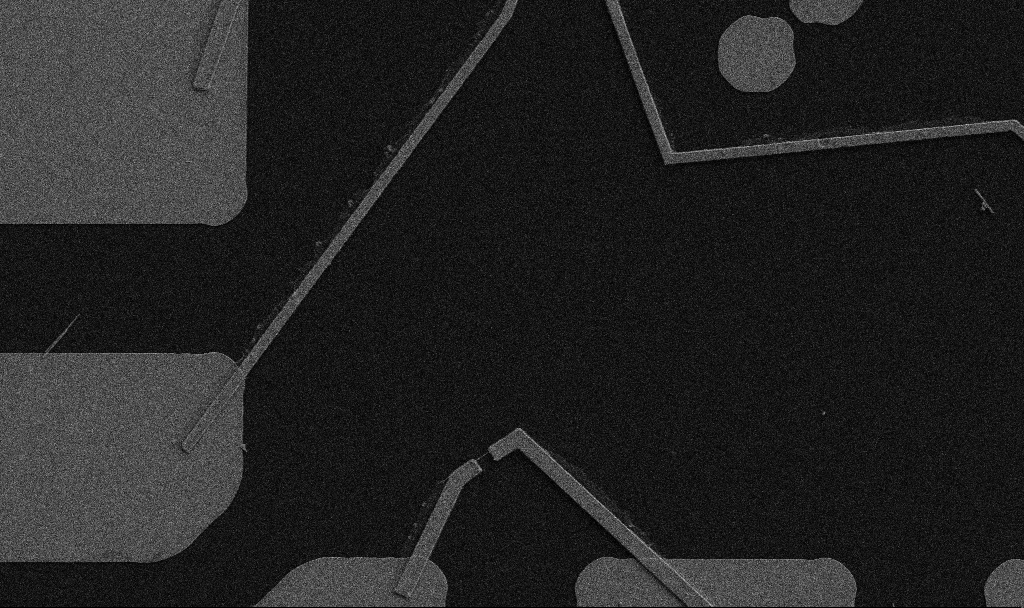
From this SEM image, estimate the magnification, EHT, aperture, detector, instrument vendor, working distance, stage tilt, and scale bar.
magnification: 5 K X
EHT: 5 kV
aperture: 30 µm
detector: SE2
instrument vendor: Zeiss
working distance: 10.7 mm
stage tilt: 0°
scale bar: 10000 nm